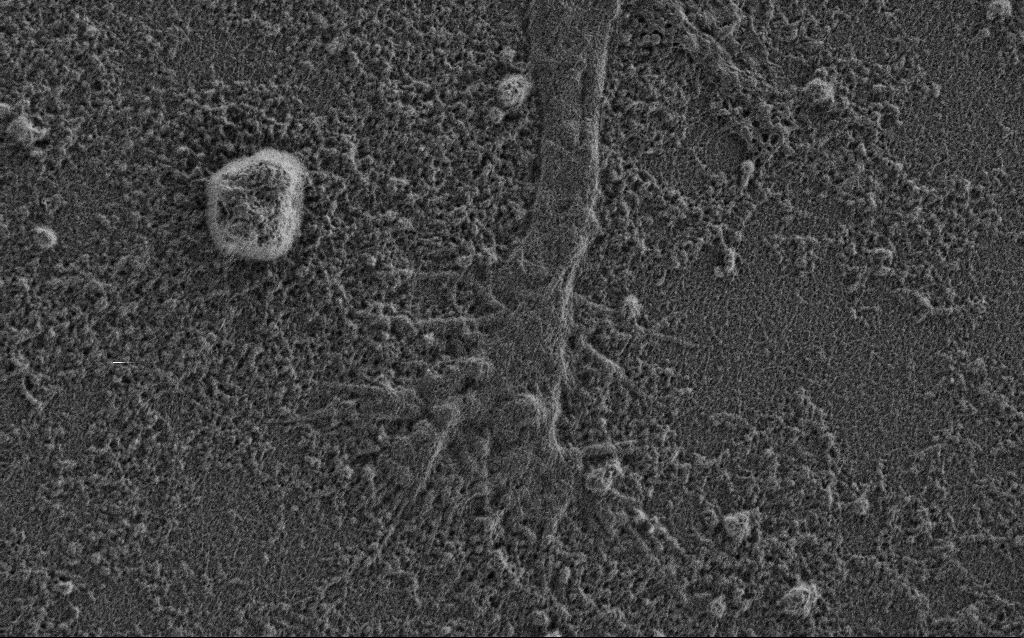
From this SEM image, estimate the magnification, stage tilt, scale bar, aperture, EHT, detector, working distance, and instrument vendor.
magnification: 10 K X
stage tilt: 0°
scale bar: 2000 nm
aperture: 30 µm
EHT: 0.9 kV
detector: SE2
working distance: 3.4 mm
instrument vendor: Zeiss